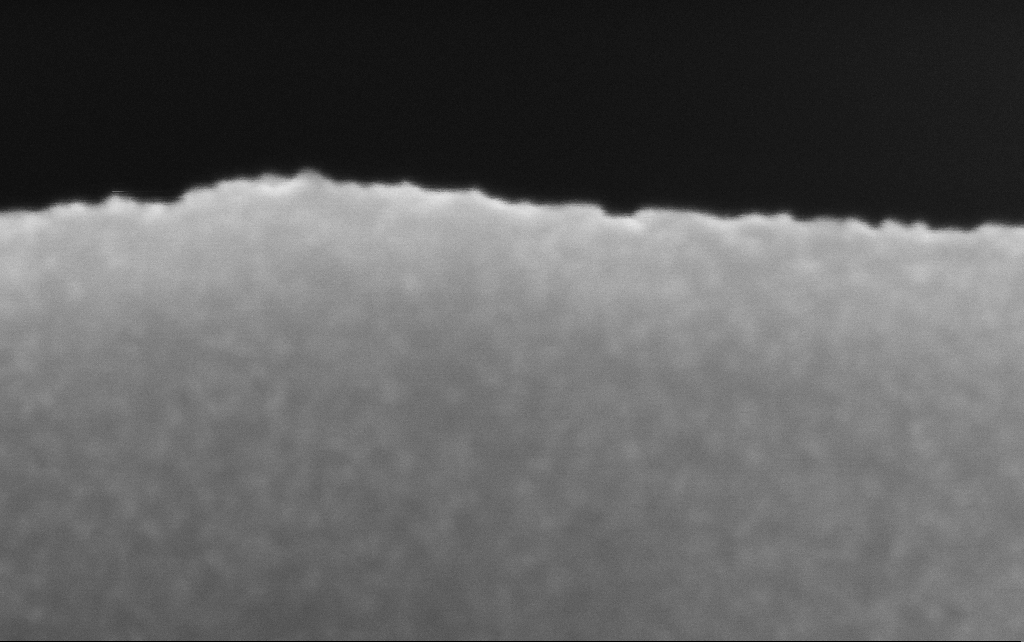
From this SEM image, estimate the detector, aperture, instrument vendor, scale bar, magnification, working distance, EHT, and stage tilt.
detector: InLens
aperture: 30 µm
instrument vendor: Zeiss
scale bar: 20 nm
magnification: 739.29 K X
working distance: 2.5 mm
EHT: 10 kV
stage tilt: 0°